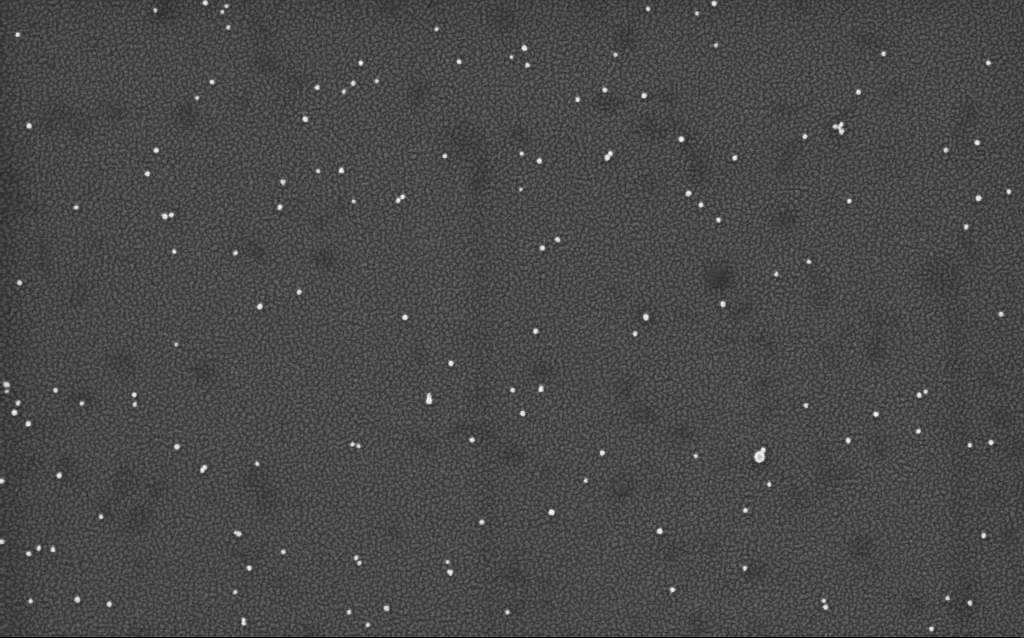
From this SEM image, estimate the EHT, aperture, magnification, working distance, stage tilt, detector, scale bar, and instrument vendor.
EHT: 2 kV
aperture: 30 µm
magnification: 100 K X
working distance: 1.7 mm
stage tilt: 0°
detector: InLens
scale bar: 200 nm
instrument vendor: Zeiss